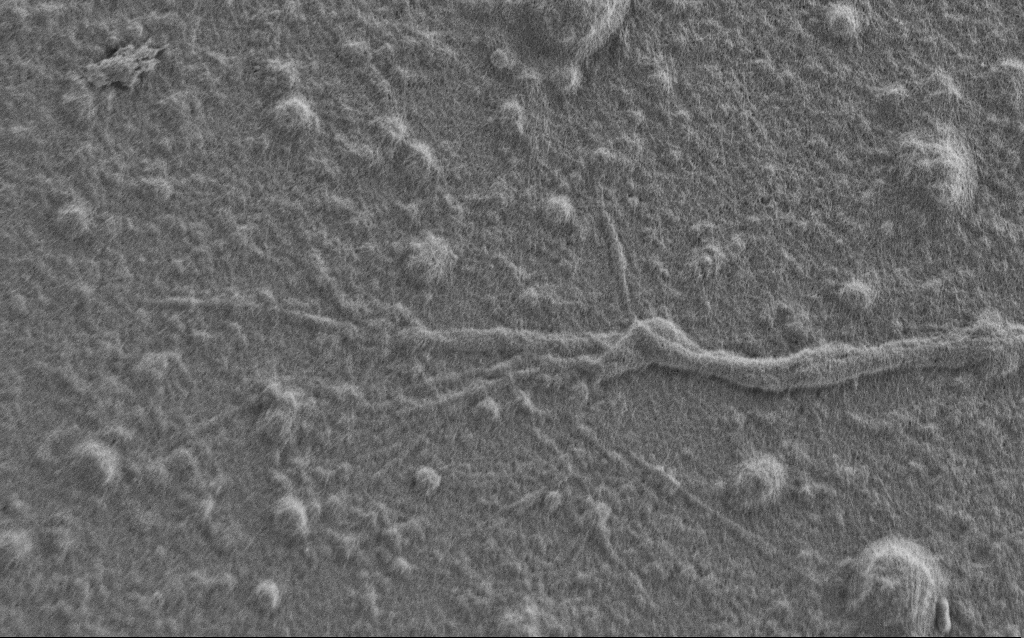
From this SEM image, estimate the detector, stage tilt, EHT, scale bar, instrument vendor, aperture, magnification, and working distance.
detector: SE2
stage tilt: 0°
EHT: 0.9 kV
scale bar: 2000 nm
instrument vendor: Zeiss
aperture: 30 µm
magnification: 7.5 K X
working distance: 7 mm